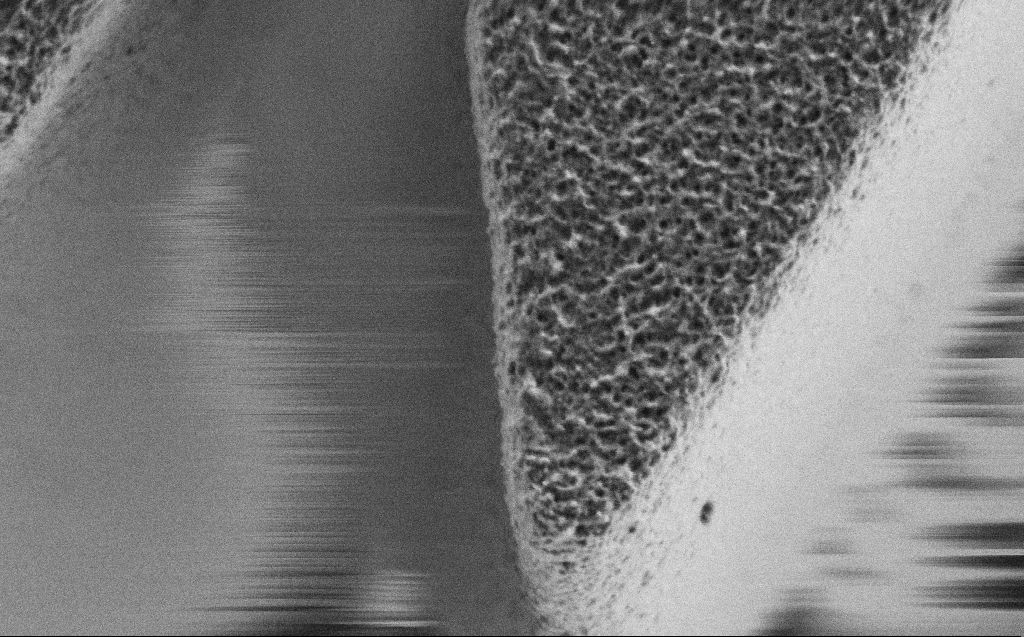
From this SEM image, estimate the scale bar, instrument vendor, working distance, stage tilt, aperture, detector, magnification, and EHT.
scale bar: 2000 nm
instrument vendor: Zeiss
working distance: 4 mm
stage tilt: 0°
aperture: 30 µm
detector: SE2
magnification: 31.86 K X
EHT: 0.9 kV